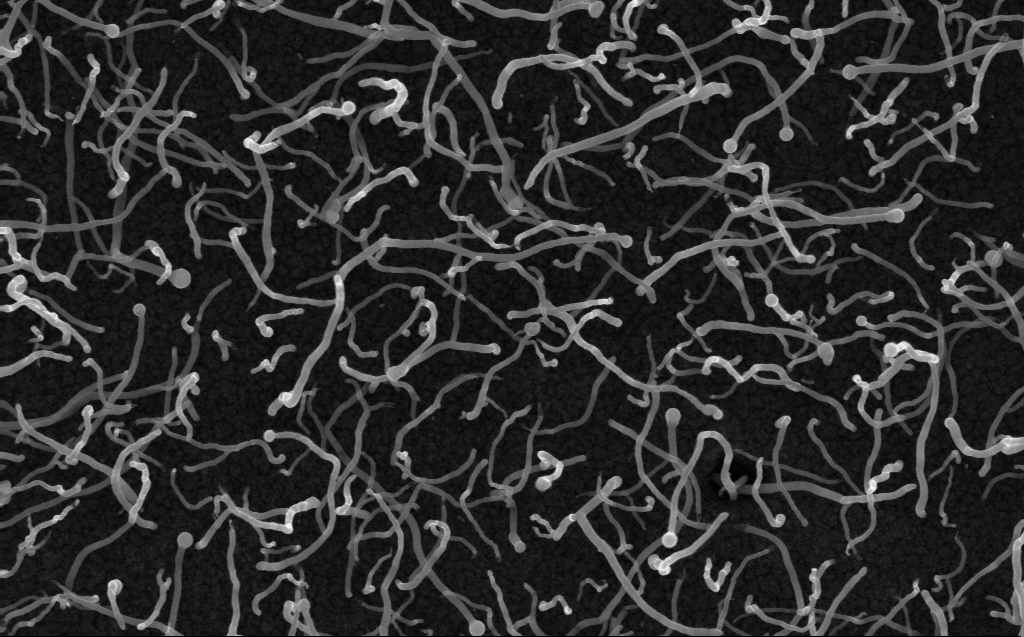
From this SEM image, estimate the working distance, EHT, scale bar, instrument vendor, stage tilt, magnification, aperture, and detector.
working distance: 3 mm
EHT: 10 kV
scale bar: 1000 nm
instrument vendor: Zeiss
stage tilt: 0°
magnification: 50 K X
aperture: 30 µm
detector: InLens